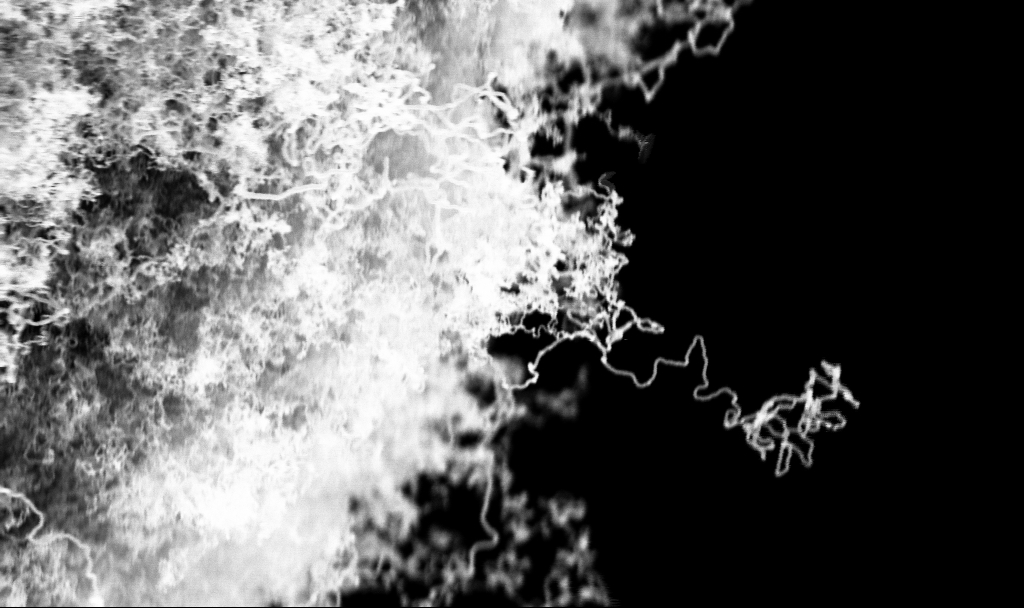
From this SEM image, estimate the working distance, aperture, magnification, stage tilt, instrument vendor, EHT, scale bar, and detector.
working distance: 2.7 mm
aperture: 30 µm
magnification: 50 K X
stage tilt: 0°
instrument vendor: Zeiss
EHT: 3 kV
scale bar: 1000 nm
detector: InLens